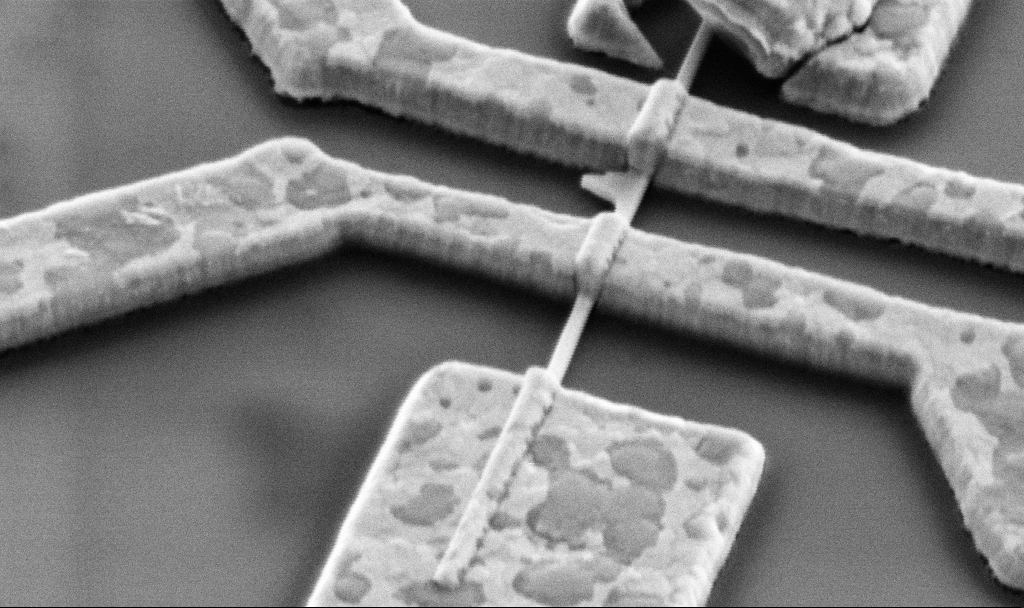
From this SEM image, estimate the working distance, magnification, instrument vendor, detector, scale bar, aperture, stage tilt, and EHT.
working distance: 14.7 mm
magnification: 60 K X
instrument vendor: Zeiss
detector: SE2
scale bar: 1000 nm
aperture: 30 µm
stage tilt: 45°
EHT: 5 kV